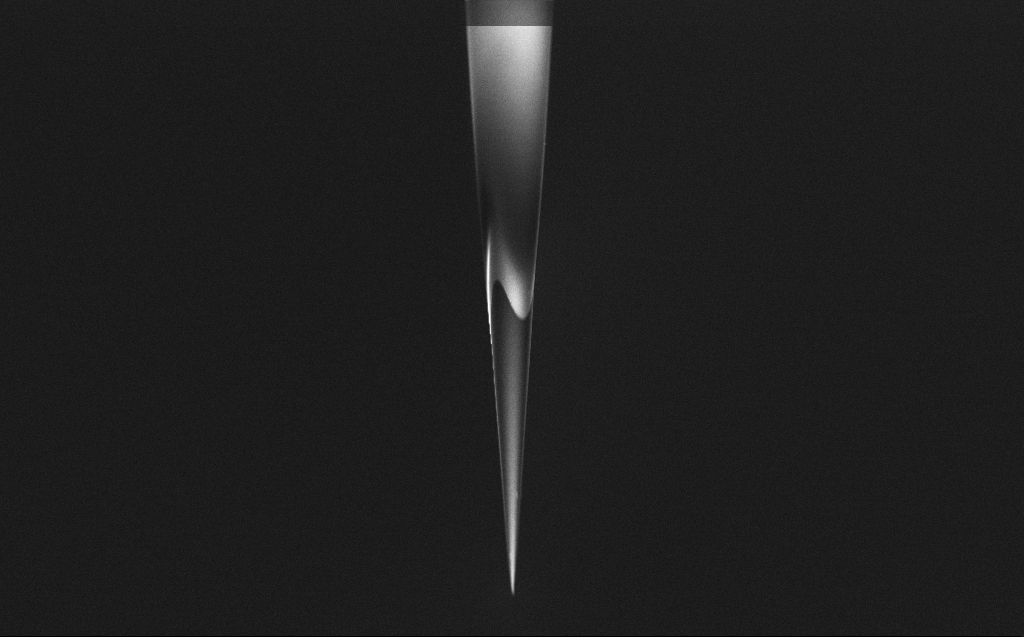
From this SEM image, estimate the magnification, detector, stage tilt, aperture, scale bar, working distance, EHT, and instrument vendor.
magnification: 2.5 K X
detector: InLens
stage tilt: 45°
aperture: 30 µm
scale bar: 10000 nm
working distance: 6 mm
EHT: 2 kV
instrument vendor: Zeiss